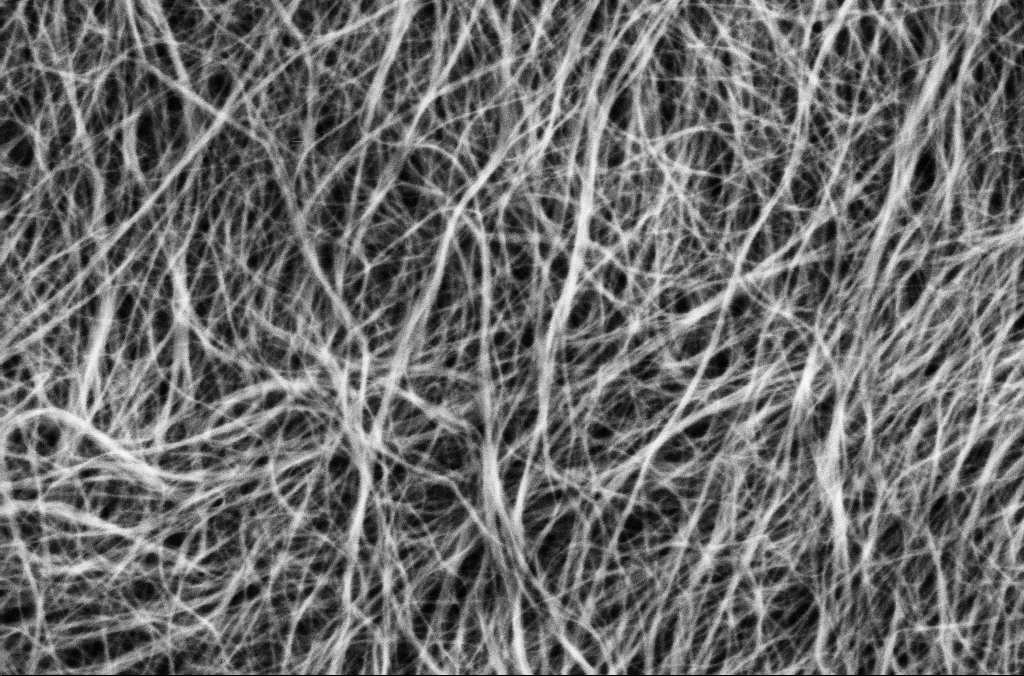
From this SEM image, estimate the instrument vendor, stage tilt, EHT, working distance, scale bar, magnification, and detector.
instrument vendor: Zeiss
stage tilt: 0°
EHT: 1.8 kV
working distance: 5.7 mm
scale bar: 200 nm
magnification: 100 K X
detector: SE2